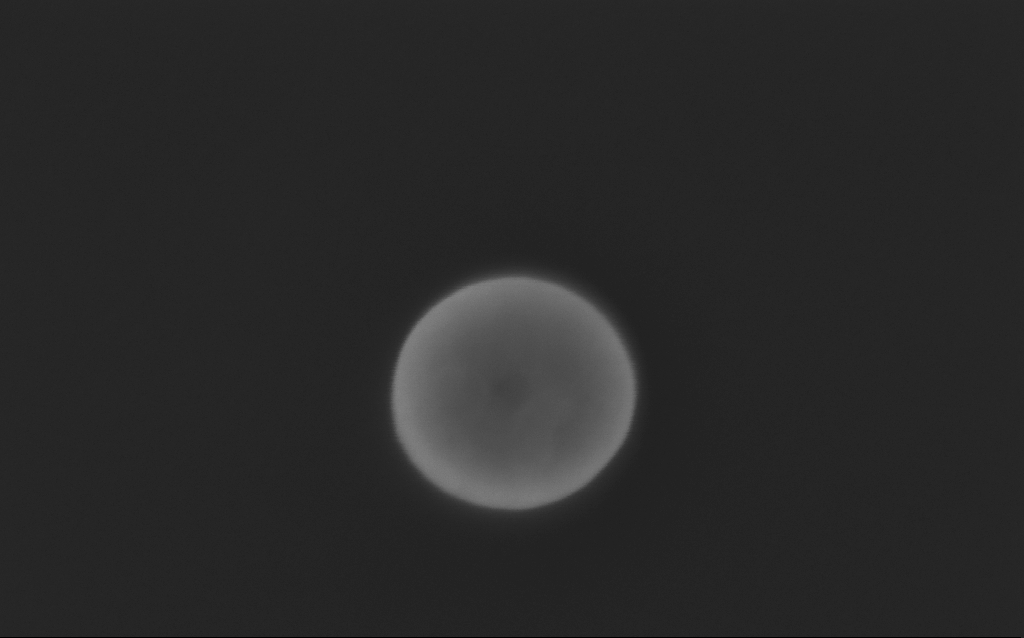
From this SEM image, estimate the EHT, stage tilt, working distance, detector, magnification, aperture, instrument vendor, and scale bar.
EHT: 5 kV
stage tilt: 0°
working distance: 3 mm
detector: InLens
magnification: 339.76 K X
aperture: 30 µm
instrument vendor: Zeiss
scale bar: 200 nm